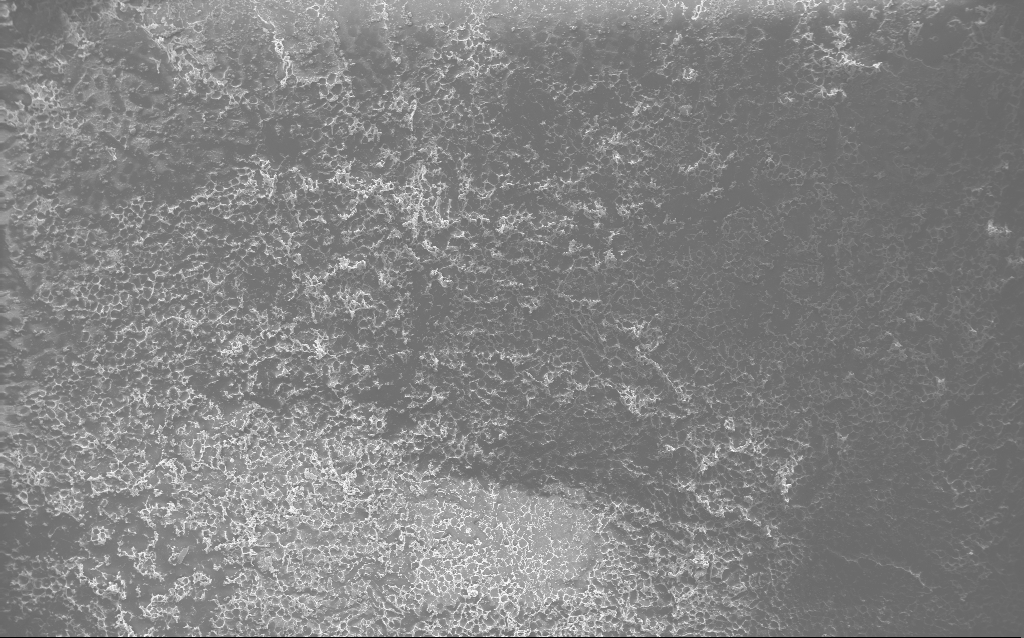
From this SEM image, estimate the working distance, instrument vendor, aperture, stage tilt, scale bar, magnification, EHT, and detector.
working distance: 2.8 mm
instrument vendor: Zeiss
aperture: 30 µm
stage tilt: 0°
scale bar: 100000 nm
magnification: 0.122 K X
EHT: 10 kV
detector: InLens